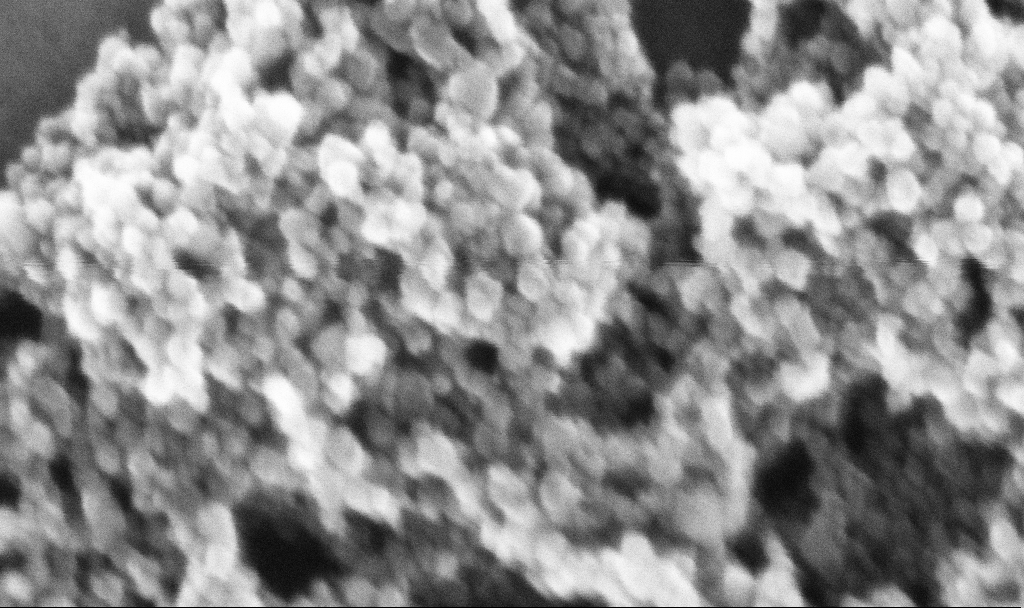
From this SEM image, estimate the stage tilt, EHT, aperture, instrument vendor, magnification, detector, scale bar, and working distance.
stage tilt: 0°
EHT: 10 kV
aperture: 30 µm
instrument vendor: Zeiss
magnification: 520.43 K X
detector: InLens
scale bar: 100 nm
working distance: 5.3 mm